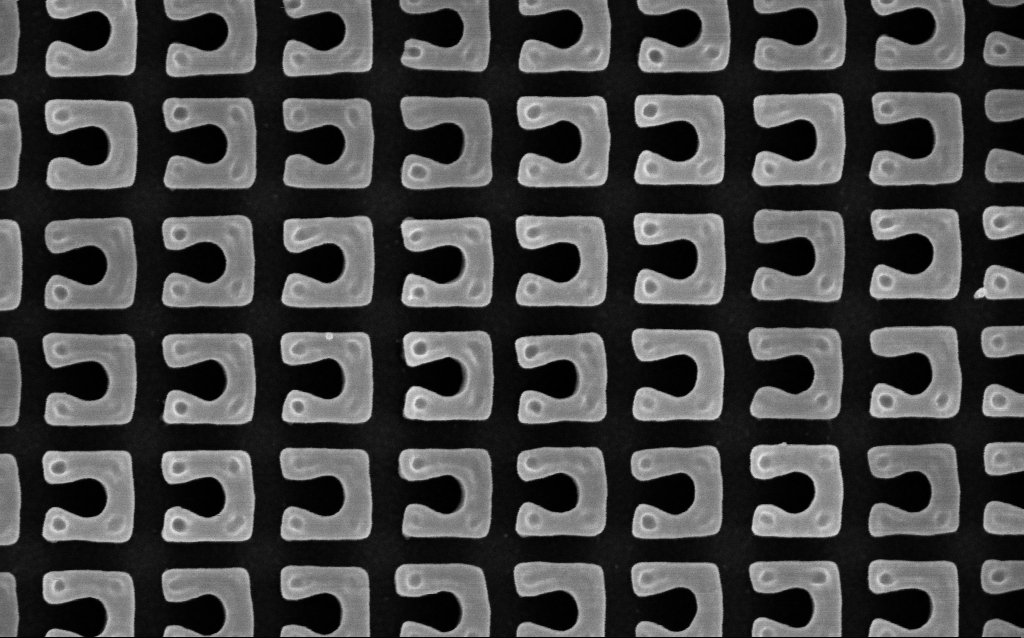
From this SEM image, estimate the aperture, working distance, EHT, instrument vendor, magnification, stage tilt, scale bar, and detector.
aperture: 30 µm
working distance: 4.3 mm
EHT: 3 kV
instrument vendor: Zeiss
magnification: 94.1 K X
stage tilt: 0°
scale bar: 200 nm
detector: InLens